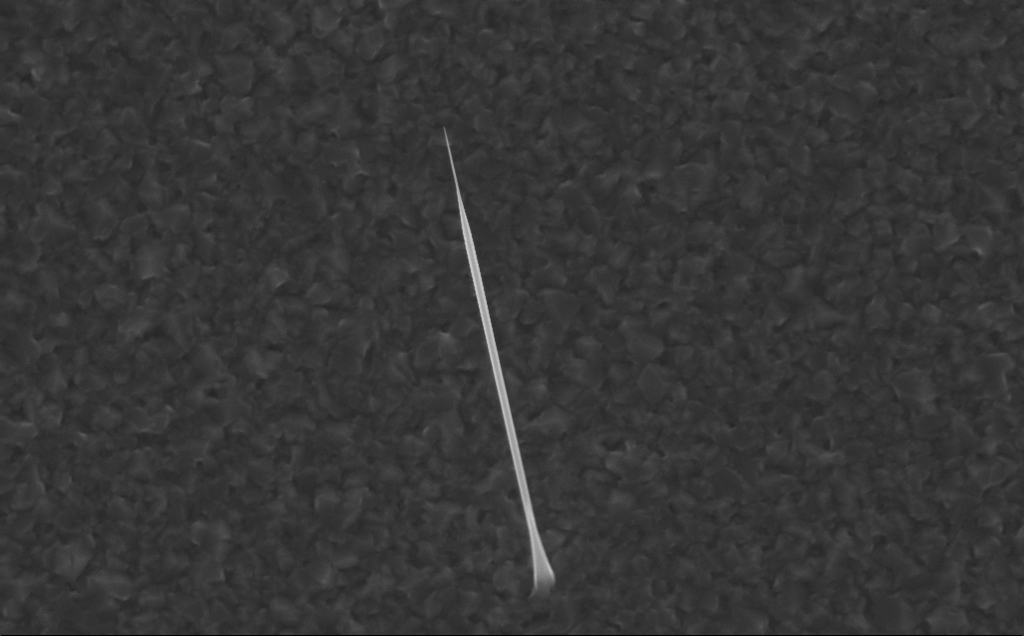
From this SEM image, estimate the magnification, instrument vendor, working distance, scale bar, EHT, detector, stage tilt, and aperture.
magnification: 22.97 K X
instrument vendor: Zeiss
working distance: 5 mm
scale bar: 2000 nm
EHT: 10 kV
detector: InLens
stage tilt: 0°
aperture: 30 µm